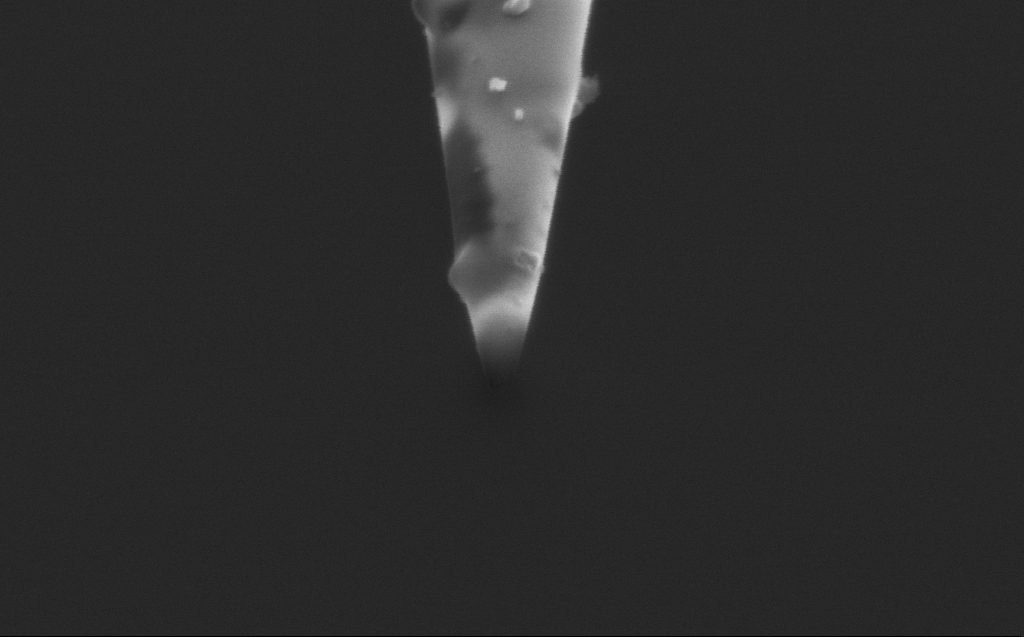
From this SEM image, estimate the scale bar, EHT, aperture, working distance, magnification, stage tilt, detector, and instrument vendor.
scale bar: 200 nm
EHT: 1.75 kV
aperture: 20 µm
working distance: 3 mm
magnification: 108.09 K X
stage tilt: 45.1°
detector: InLens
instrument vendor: Zeiss